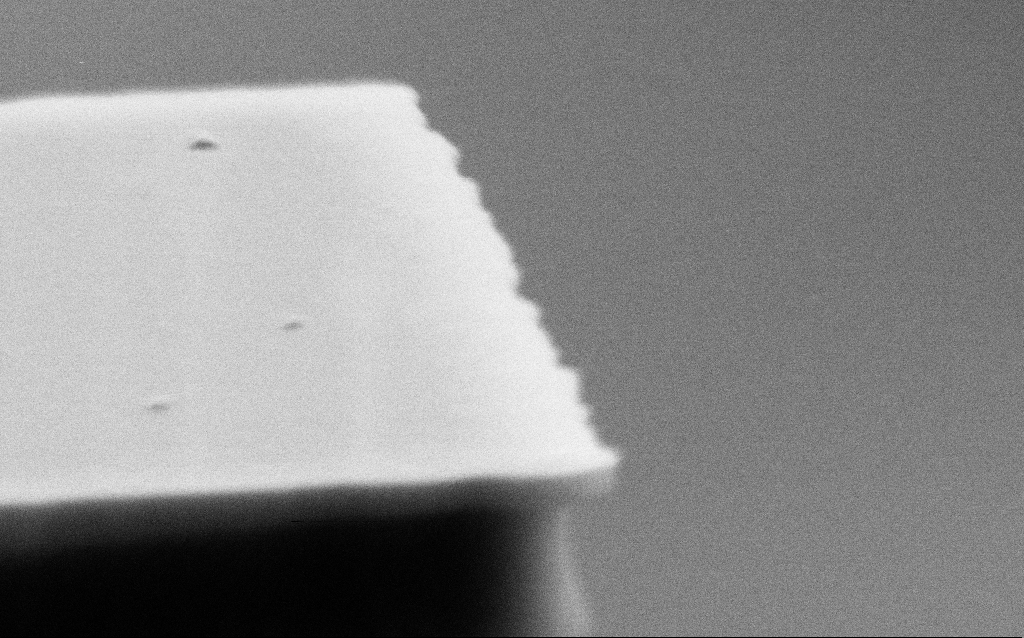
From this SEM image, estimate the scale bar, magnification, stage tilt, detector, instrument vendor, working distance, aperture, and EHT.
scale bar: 200 nm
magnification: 160.58 K X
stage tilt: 70°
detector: SE2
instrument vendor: Zeiss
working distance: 4 mm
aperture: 30 µm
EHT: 5 kV